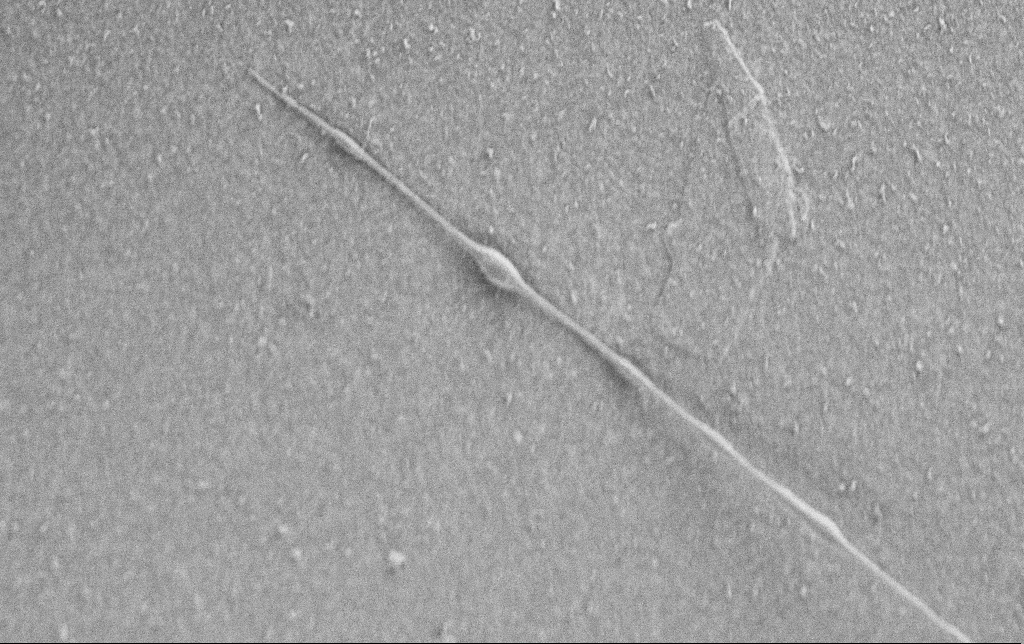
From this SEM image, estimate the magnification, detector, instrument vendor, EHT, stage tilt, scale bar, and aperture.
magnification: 10 K X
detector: SE2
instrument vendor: Zeiss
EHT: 0.9 kV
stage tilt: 0°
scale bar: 2000 nm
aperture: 30 µm